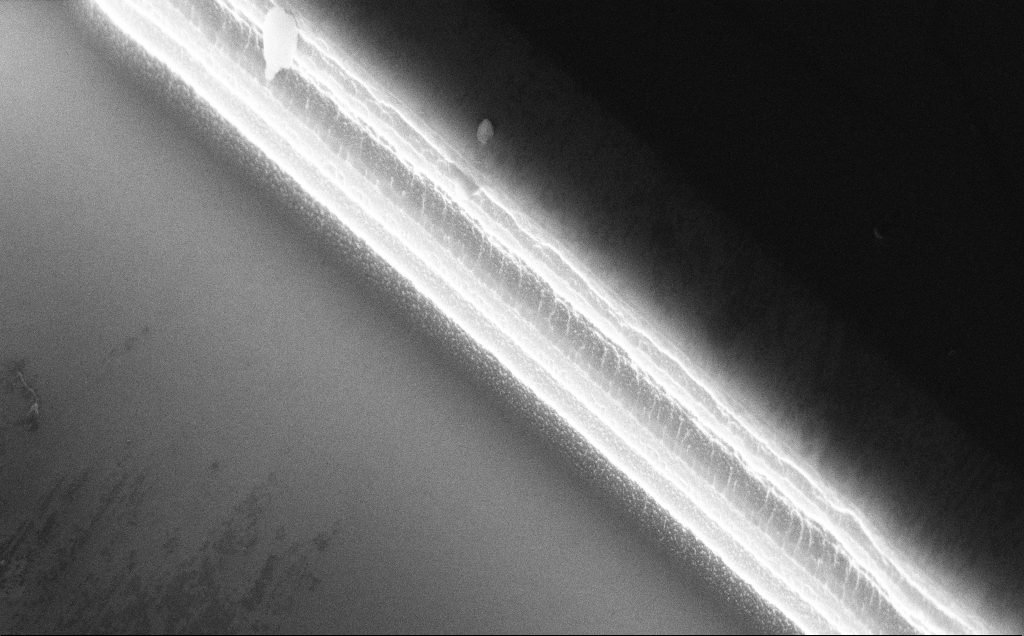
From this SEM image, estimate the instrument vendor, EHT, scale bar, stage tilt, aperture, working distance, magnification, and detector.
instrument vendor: Zeiss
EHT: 10 kV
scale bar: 2000 nm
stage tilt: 50°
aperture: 30 µm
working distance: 9 mm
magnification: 24.87 K X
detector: InLens